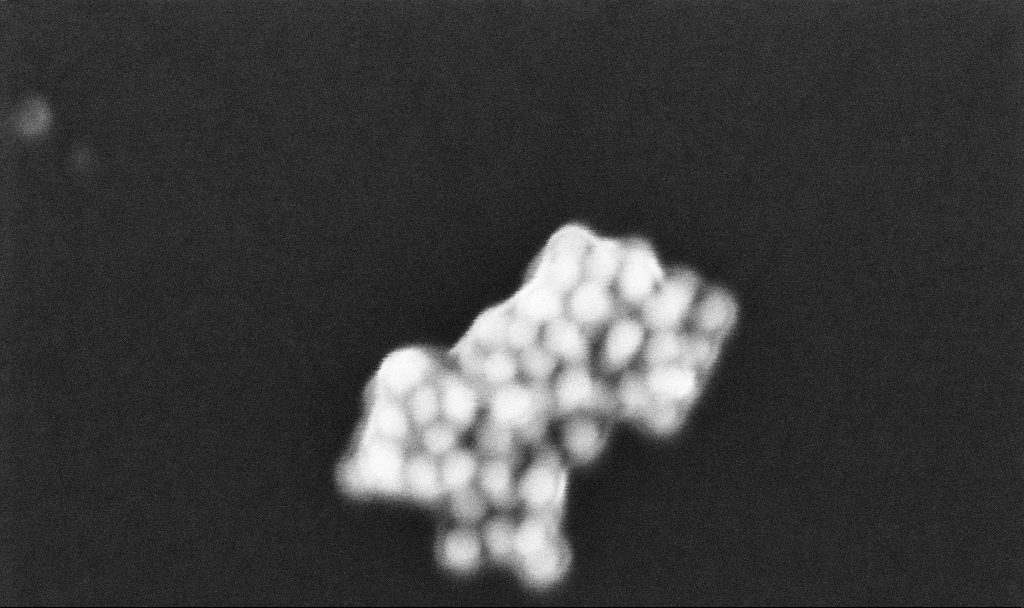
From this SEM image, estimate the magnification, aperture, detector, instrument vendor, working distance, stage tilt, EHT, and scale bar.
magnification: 667.82 K X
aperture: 30 µm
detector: InLens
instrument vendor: Zeiss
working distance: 3.2 mm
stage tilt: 0°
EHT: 10 kV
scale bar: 100 nm